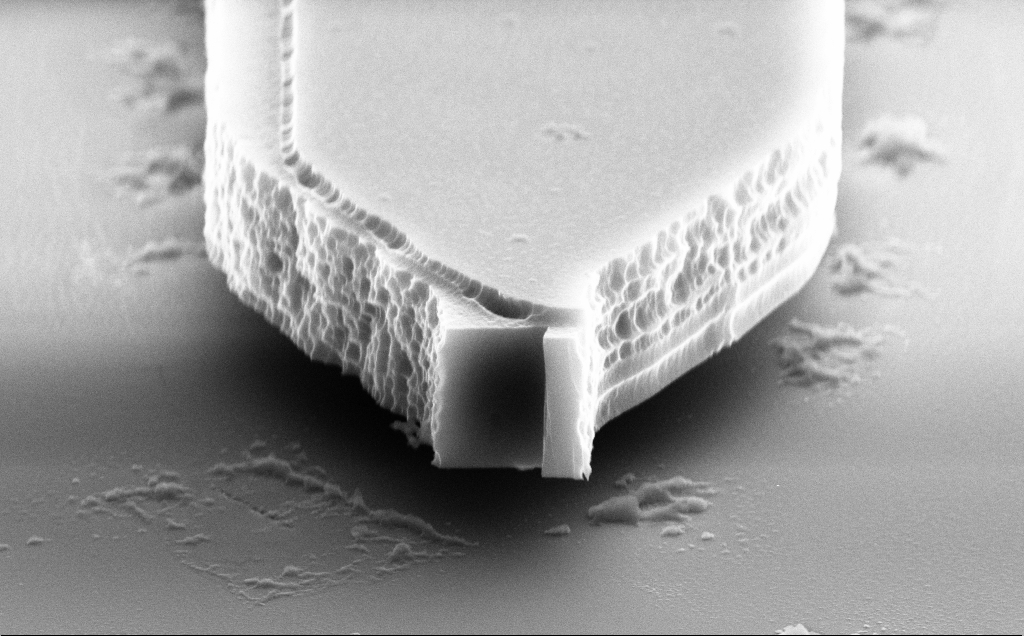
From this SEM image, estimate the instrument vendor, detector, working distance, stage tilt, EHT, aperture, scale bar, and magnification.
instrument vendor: Zeiss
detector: SE2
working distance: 10 mm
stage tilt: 70°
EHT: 10 kV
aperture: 30 µm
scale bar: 1000 nm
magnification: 24.78 K X